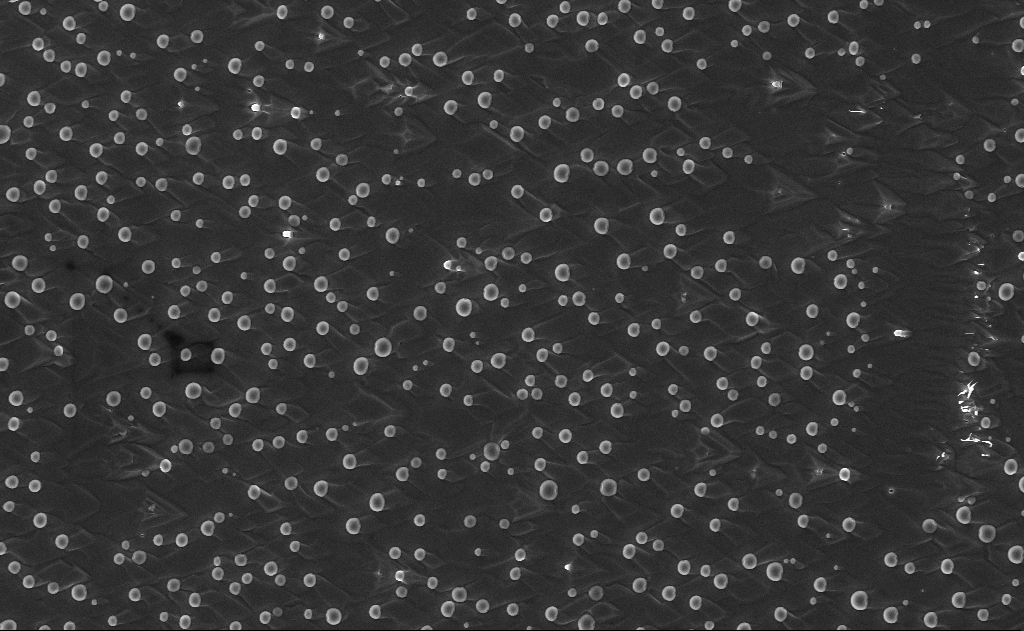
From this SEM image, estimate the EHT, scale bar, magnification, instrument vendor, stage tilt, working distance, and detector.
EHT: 10 kV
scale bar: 10000 nm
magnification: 5 K X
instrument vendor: Zeiss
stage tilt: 0°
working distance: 10 mm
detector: InLens